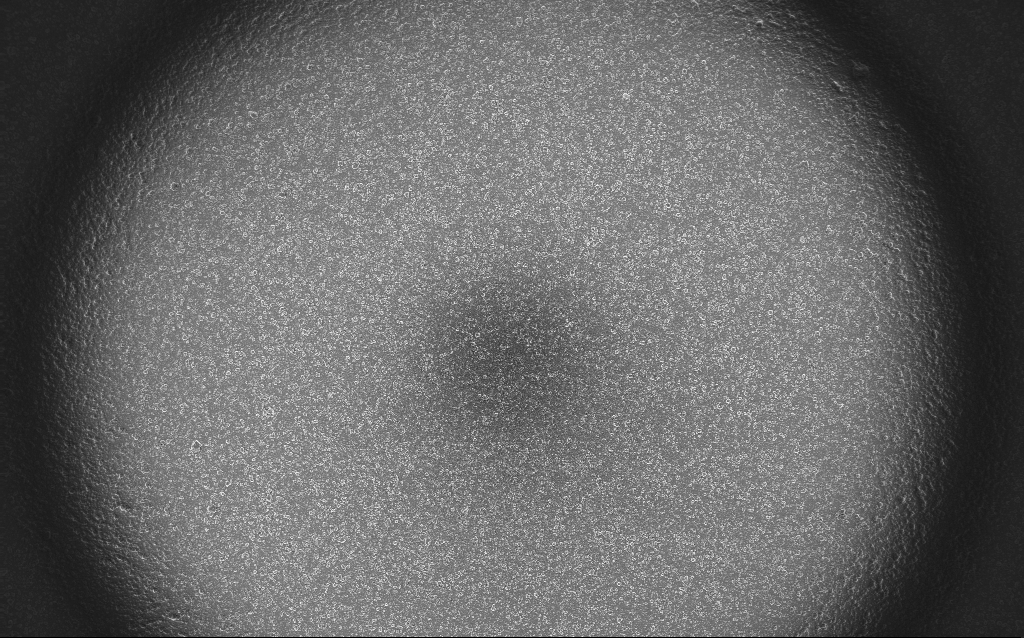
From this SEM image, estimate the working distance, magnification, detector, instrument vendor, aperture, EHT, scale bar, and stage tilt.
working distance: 2.7 mm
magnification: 0.122 K X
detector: InLens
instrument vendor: Zeiss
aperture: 30 µm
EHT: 10 kV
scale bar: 100000 nm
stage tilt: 0°